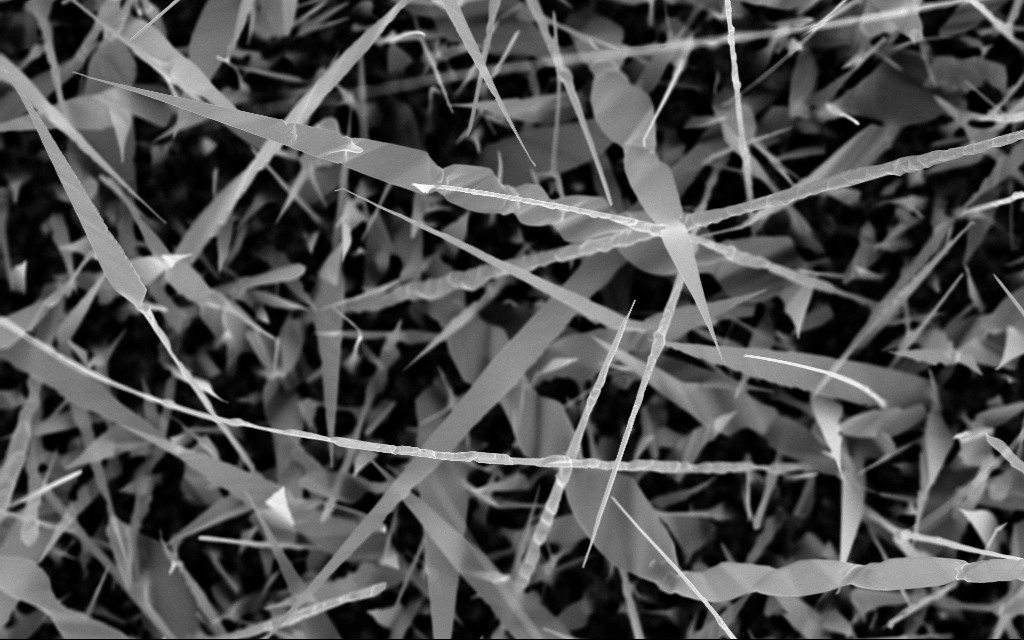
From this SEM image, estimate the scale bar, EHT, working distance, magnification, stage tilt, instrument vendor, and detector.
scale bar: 2000 nm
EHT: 10 kV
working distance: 6 mm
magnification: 21.26 K X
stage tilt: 0°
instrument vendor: Zeiss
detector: InLens